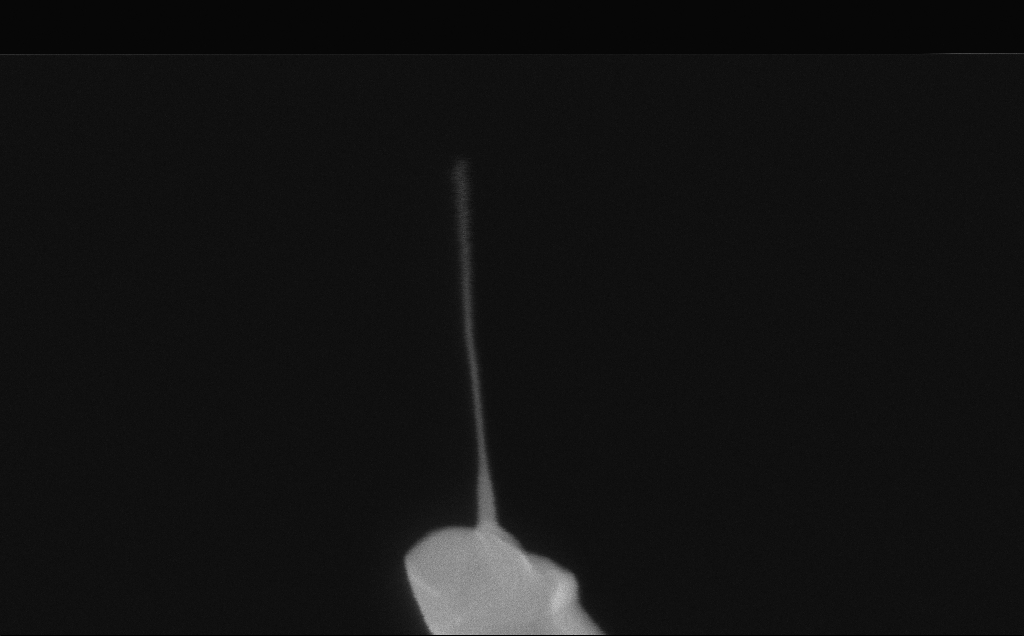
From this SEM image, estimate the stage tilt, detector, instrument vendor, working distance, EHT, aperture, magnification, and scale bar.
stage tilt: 0°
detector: InLens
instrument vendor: Zeiss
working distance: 6 mm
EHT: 10 kV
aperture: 30 µm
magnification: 257.27 K X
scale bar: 200 nm